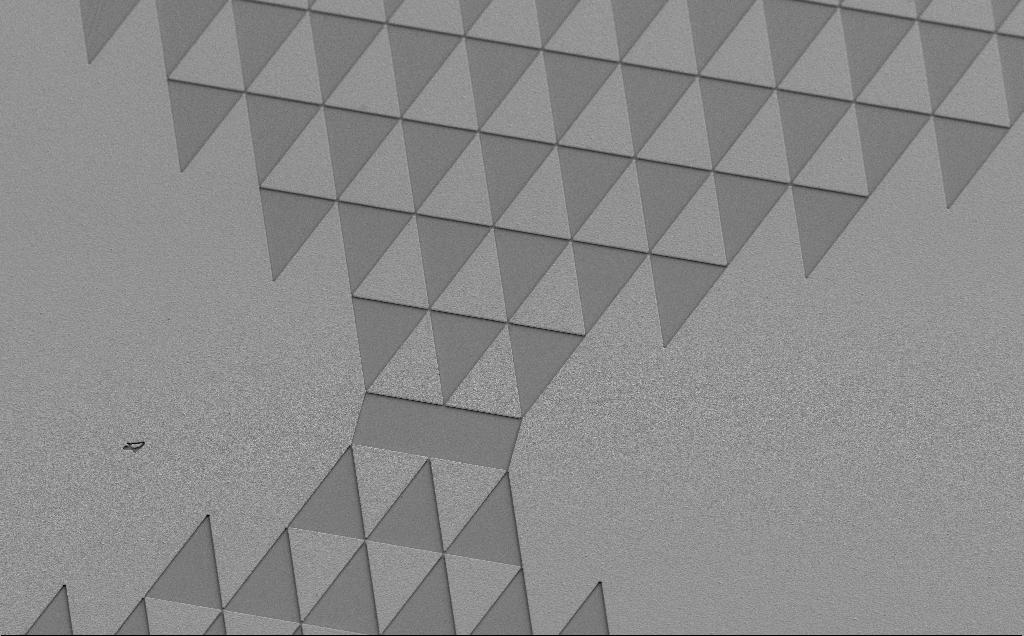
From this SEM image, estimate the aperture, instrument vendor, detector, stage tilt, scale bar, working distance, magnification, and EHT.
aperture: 30 µm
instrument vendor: Zeiss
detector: SE2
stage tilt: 35°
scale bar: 100000 nm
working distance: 13 mm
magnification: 0.488 K X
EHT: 5 kV